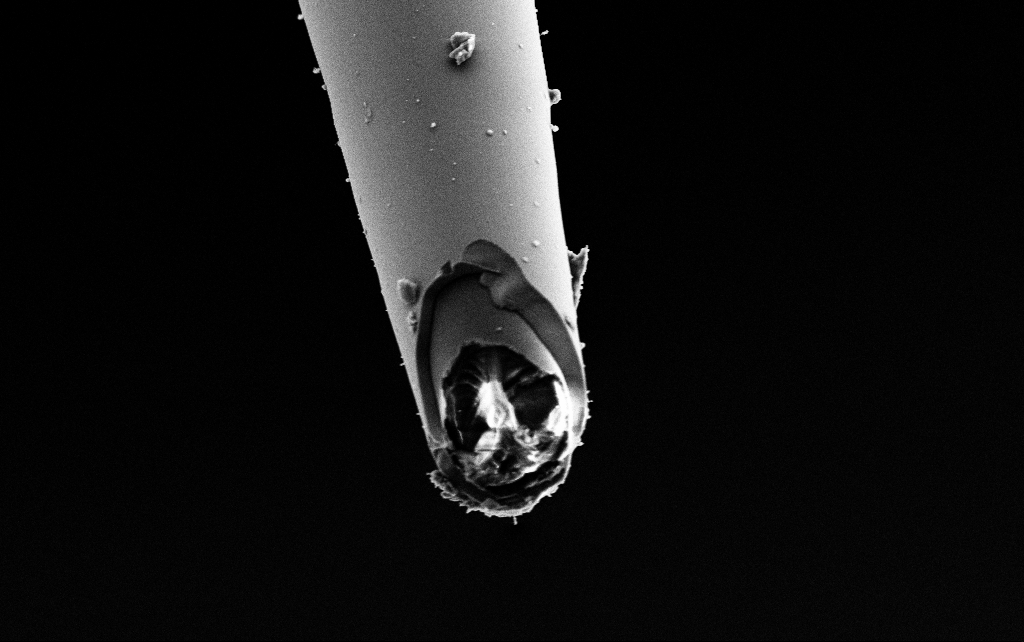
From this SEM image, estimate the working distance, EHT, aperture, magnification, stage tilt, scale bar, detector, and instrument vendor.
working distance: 7.9 mm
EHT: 3 kV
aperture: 30 µm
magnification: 10 K X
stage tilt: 45°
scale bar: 2000 nm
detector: SE2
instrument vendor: Zeiss